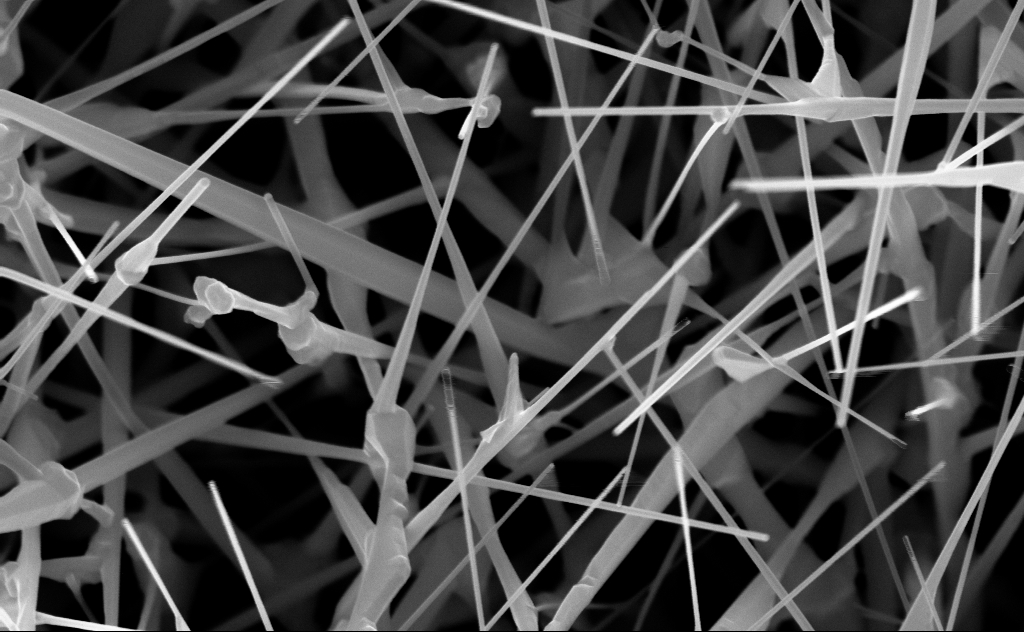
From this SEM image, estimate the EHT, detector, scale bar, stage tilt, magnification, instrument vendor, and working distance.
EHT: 10 kV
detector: InLens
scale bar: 200 nm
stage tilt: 0°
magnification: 80 K X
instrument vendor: Zeiss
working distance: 6 mm